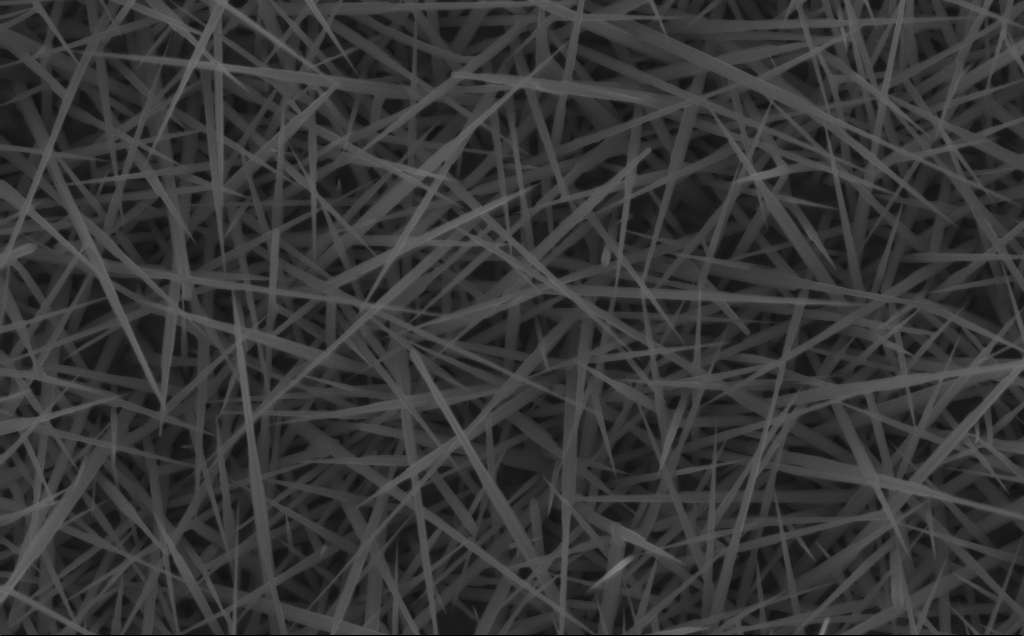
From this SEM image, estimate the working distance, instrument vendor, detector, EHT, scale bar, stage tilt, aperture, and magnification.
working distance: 4 mm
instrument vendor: Zeiss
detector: InLens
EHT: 10 kV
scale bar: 1000 nm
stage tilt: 0°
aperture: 30 µm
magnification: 40 K X